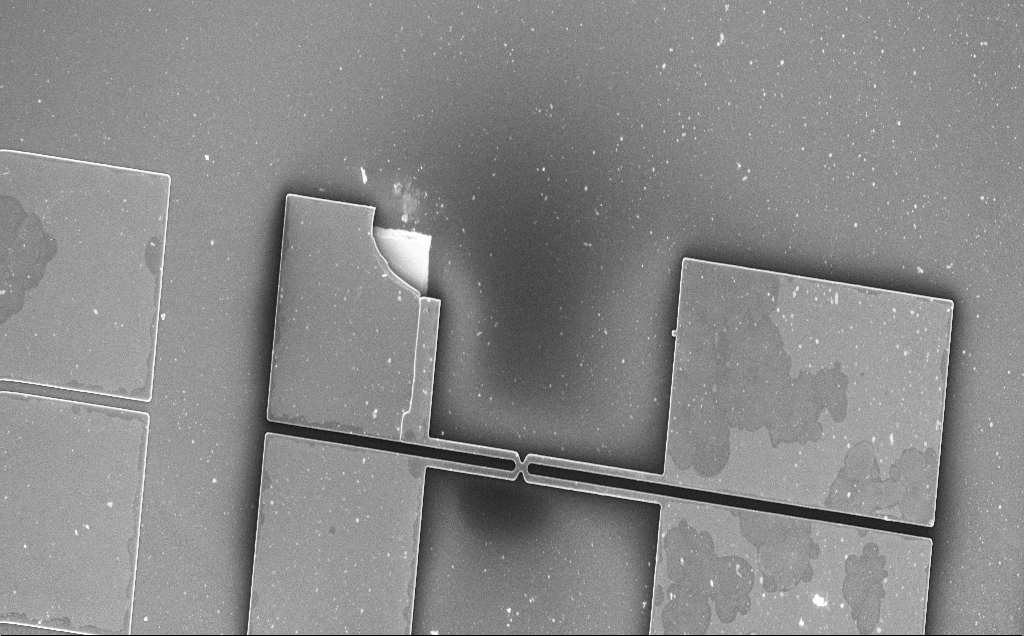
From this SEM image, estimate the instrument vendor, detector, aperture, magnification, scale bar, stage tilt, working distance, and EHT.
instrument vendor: Zeiss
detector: InLens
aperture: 30 µm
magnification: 0.291 K X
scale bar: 100000 nm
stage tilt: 0°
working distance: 4 mm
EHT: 15 kV